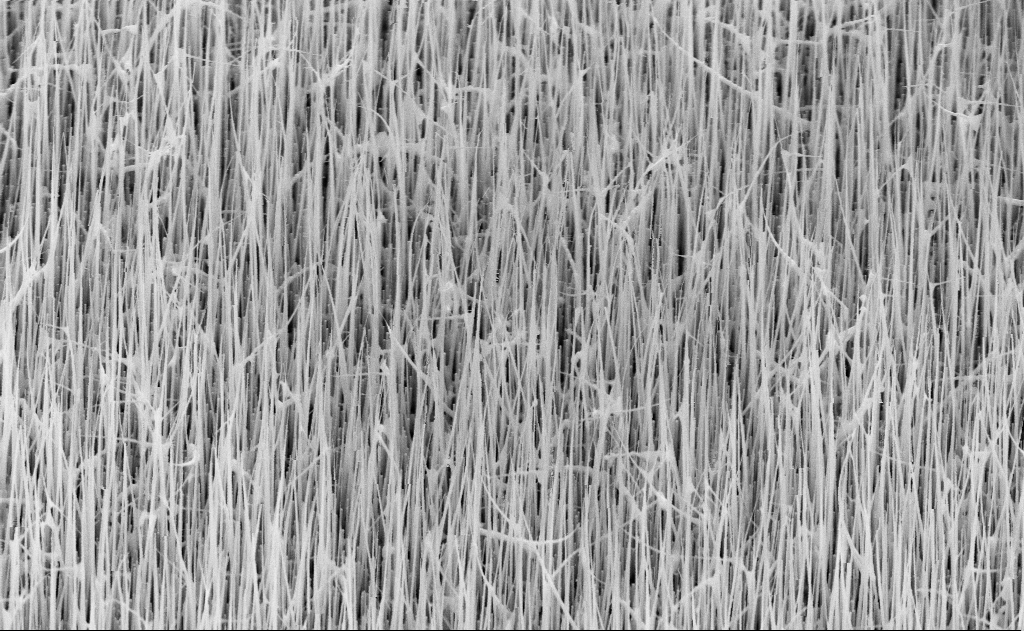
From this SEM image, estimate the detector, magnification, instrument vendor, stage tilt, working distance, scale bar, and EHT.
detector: SE2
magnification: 20 K X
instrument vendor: Zeiss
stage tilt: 45°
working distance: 16 mm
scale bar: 1000 nm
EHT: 10 kV